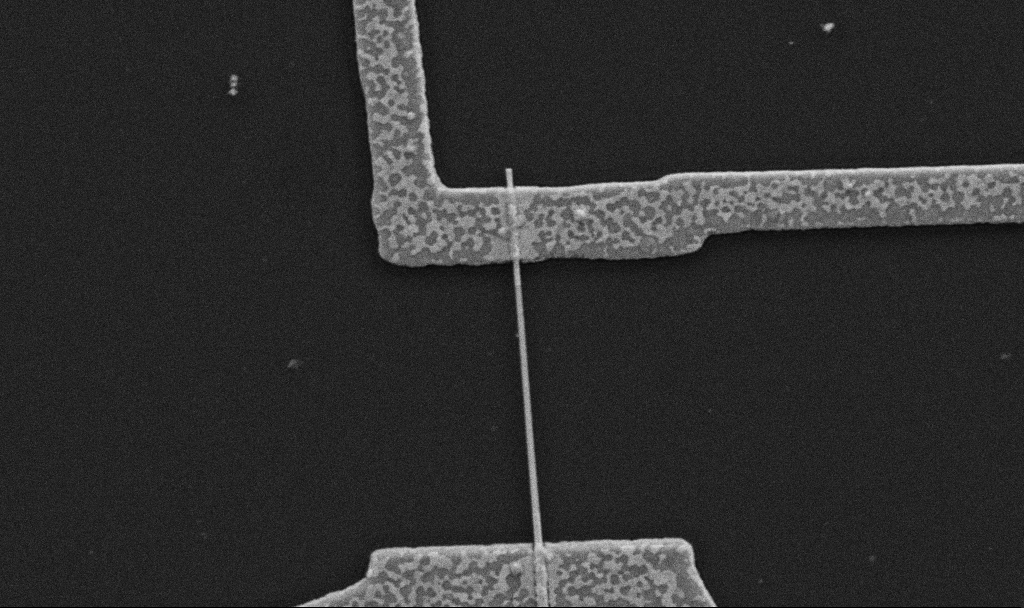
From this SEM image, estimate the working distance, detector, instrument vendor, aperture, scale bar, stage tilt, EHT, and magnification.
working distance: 10.7 mm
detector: SE2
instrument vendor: Zeiss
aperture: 30 µm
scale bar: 1000 nm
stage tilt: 0°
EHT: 5 kV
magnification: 30 K X